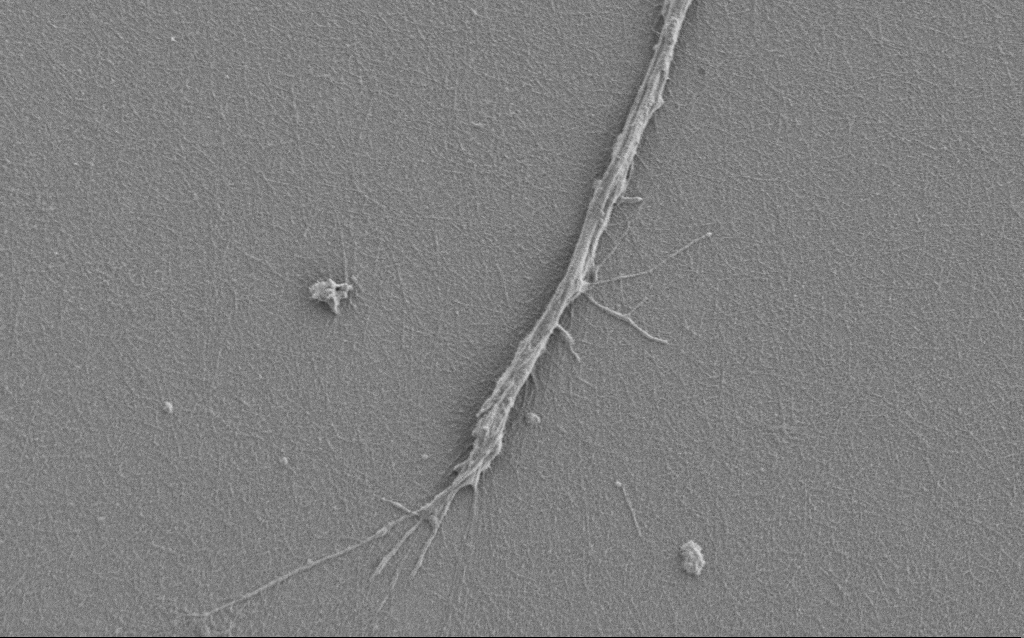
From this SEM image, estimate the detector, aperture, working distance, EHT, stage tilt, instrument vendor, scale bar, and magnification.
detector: SE2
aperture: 30 µm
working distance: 6 mm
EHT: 1 kV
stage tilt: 0°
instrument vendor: Zeiss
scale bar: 2000 nm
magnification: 7.5 K X